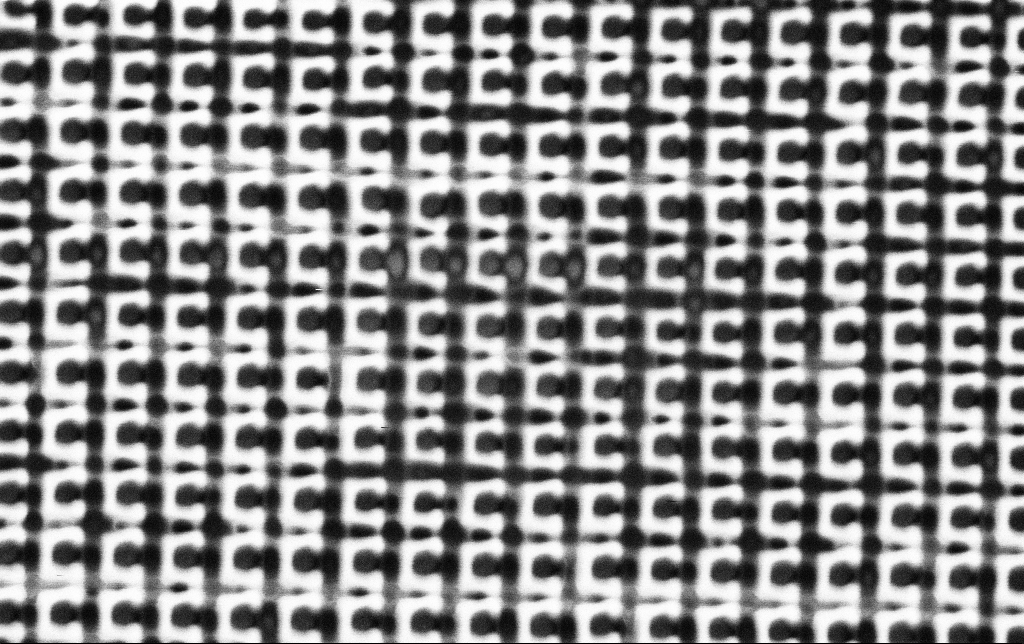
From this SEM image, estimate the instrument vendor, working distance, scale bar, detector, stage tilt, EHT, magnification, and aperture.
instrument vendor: Zeiss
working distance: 8.1 mm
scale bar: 1000 nm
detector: SE2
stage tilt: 0°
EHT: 1.5 kV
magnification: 47.62 K X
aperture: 30 µm